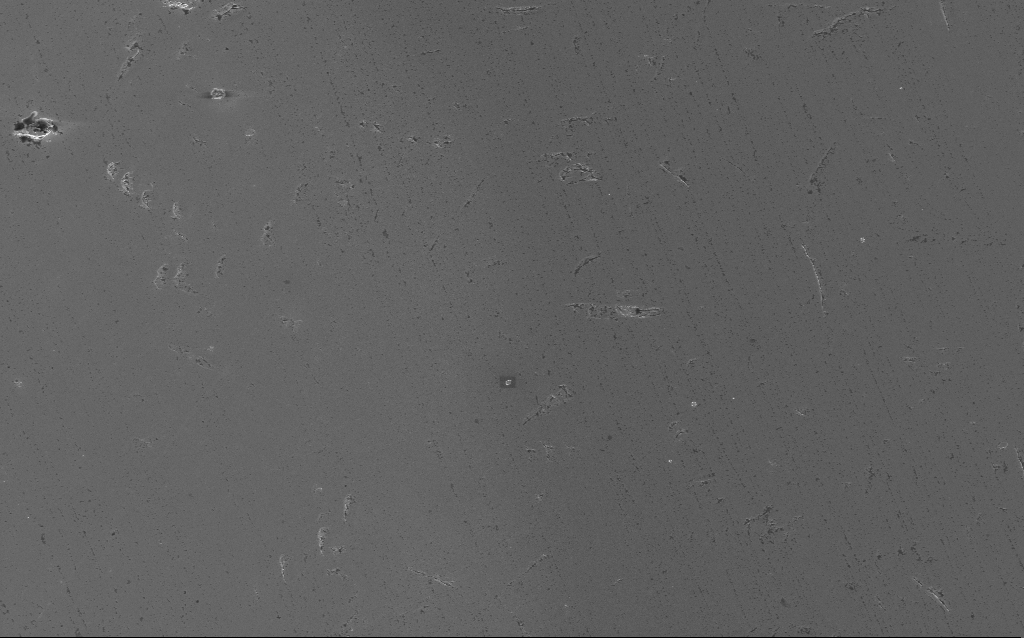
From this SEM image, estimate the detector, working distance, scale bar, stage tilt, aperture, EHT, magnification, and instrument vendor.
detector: InLens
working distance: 5 mm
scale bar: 20000 nm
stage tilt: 0°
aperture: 30 µm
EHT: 5 kV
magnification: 2.05 K X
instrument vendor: Zeiss